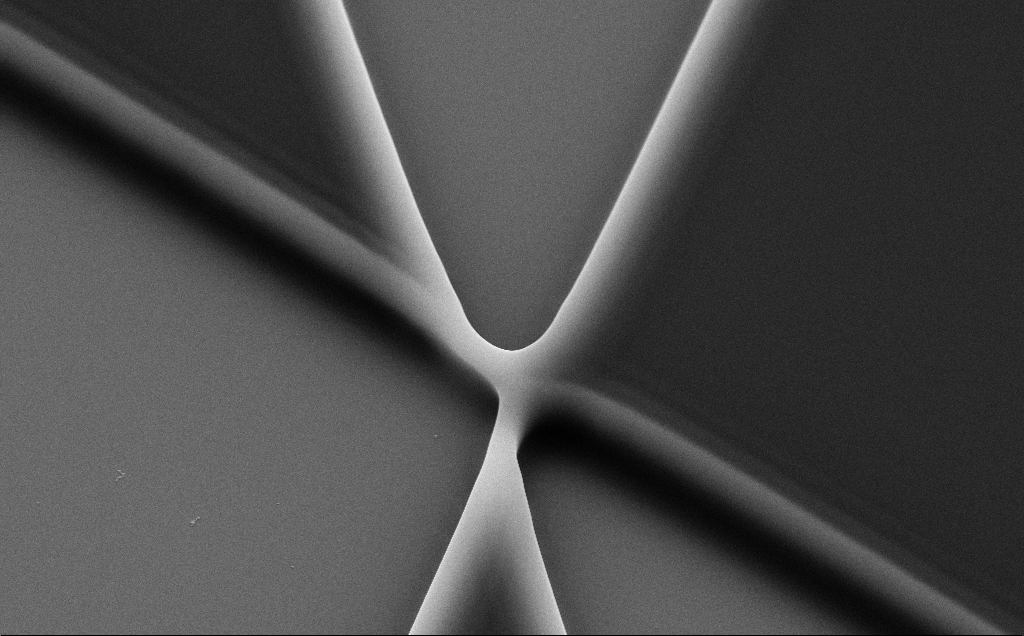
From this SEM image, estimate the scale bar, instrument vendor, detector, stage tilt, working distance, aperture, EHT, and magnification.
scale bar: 1000 nm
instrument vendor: Zeiss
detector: SE2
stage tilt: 35°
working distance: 8 mm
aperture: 30 µm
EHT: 10 kV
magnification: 15.74 K X